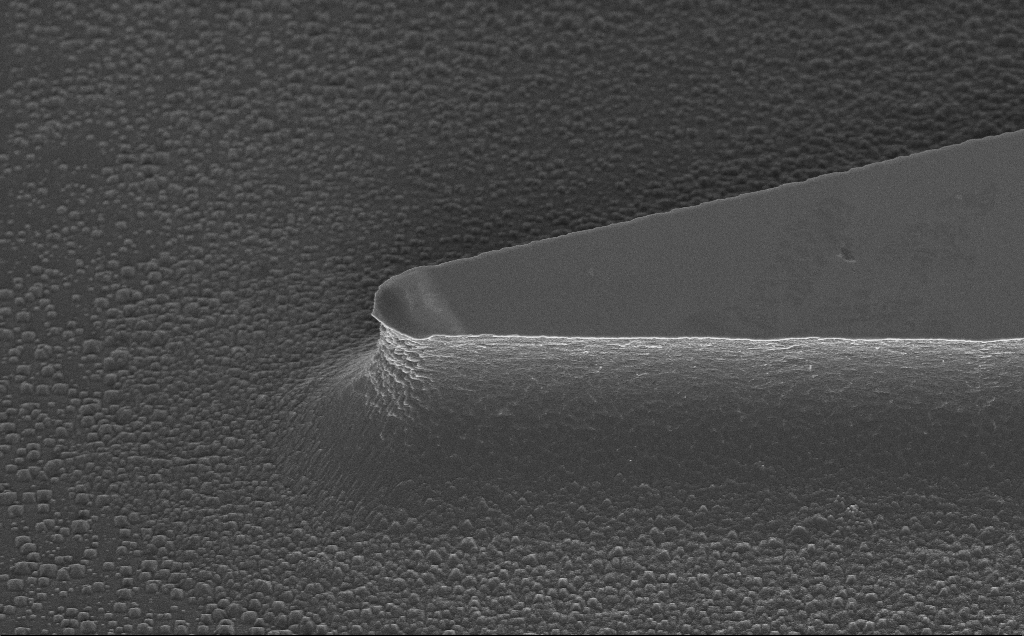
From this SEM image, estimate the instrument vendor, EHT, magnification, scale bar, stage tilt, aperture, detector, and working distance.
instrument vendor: Zeiss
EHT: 5 kV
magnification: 5.11 K X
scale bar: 10000 nm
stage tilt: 45°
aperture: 30 µm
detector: InLens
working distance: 13 mm